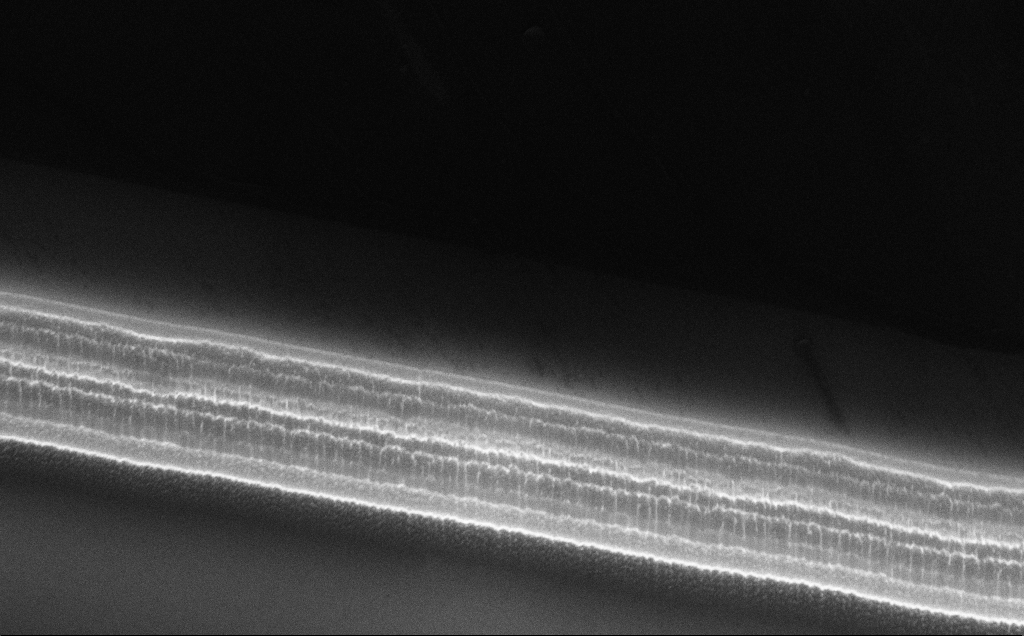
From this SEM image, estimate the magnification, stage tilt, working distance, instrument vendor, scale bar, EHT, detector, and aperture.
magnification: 21.8 K X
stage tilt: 50°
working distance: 10 mm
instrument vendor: Zeiss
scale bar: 1000 nm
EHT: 10 kV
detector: InLens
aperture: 30 µm